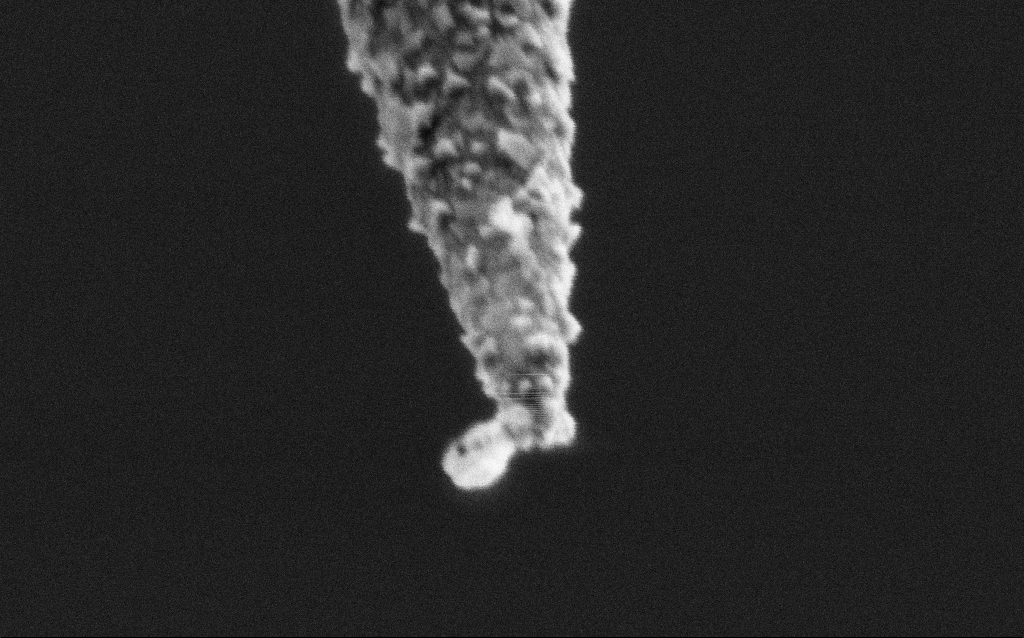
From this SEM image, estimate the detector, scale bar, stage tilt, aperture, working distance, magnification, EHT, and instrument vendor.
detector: SE2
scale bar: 100 nm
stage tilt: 45°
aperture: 30 µm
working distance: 7.6 mm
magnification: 150 K X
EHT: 2 kV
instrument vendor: Zeiss